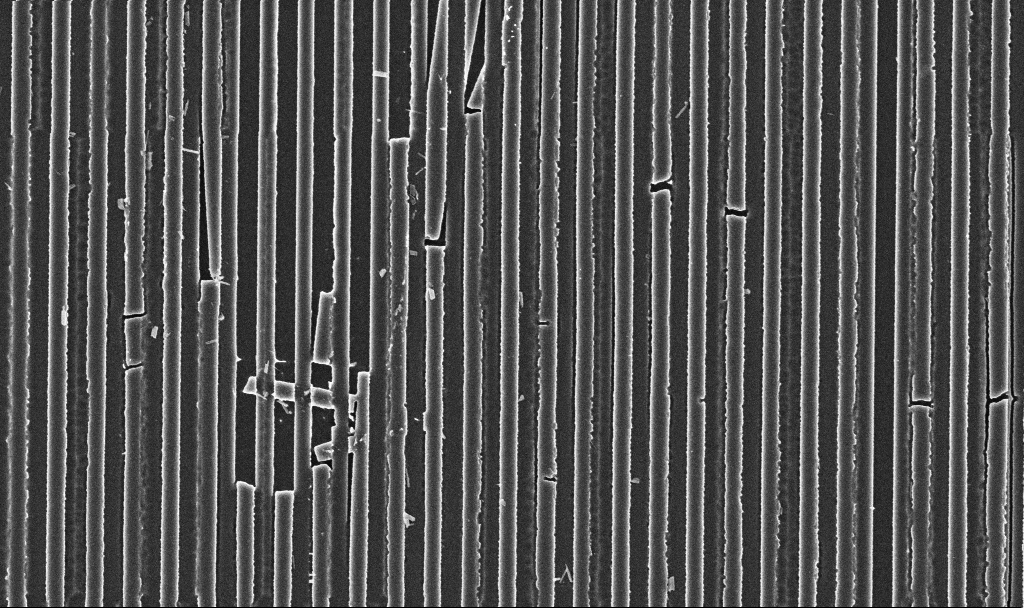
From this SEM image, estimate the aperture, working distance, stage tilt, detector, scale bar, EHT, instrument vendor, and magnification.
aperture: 30 µm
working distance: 2.9 mm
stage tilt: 0°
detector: InLens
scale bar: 2000 nm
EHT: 3 kV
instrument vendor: Zeiss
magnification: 21.09 K X